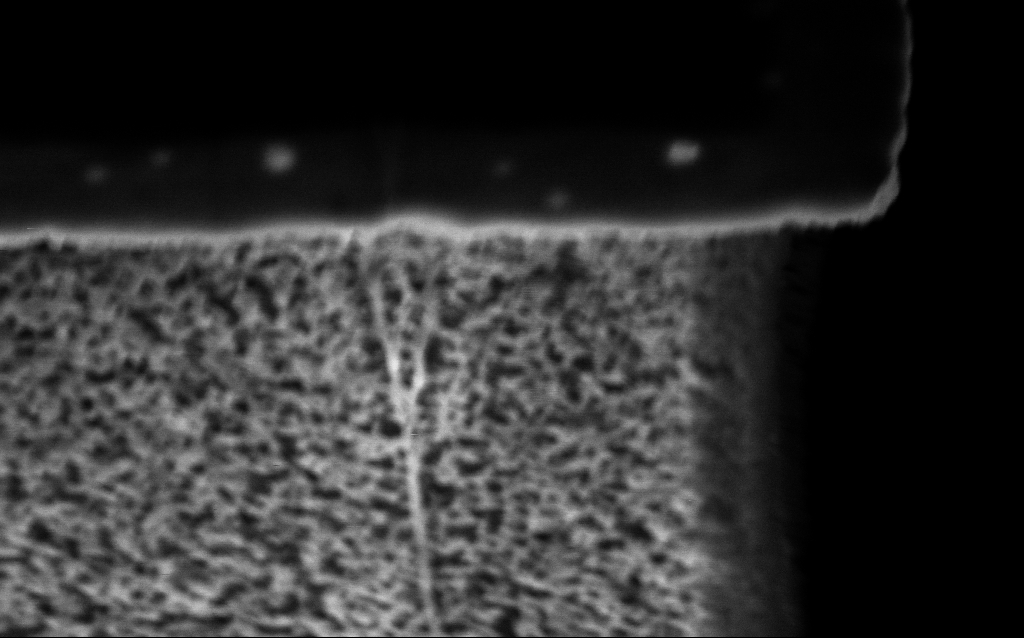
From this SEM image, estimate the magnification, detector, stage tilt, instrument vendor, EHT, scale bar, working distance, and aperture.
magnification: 141.75 K X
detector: InLens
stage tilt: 45°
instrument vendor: Zeiss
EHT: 3 kV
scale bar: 100 nm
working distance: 6.2 mm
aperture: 30 µm